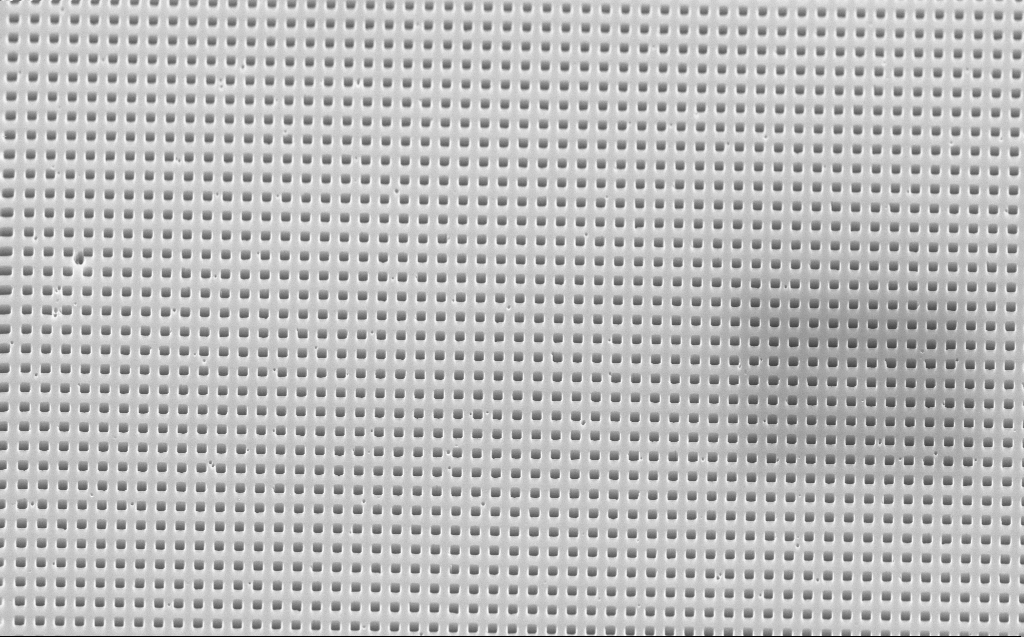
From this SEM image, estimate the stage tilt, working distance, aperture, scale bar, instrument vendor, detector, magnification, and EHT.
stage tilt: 45°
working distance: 6 mm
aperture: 30 µm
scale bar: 1000 nm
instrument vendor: Zeiss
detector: InLens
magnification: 14.61 K X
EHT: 10 kV